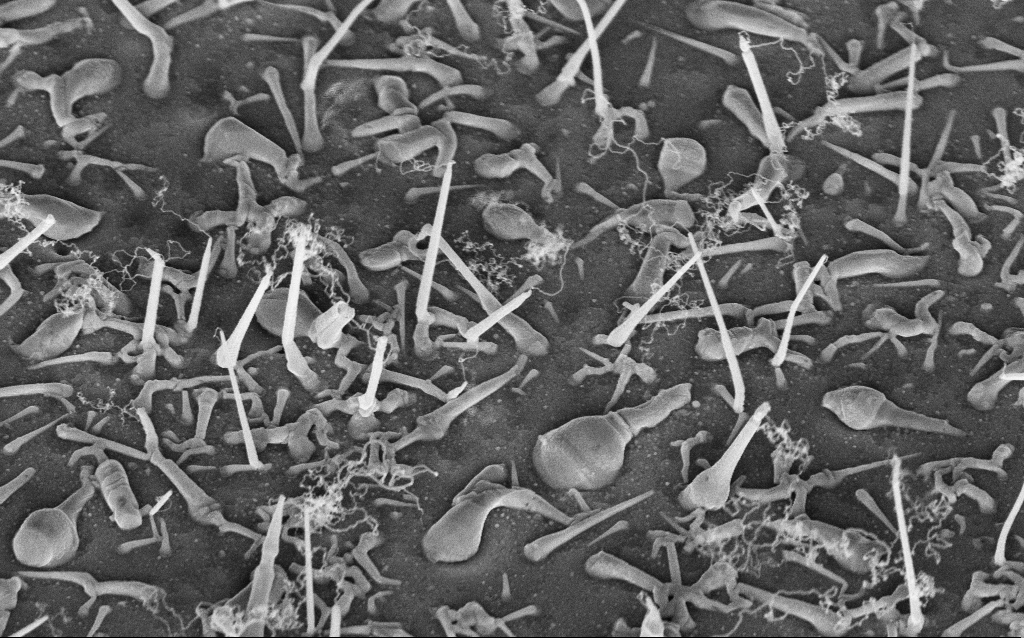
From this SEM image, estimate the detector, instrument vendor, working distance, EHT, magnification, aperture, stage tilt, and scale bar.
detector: InLens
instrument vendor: Zeiss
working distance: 8 mm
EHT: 5 kV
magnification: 34.34 K X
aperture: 30 µm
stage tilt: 42°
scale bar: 2000 nm